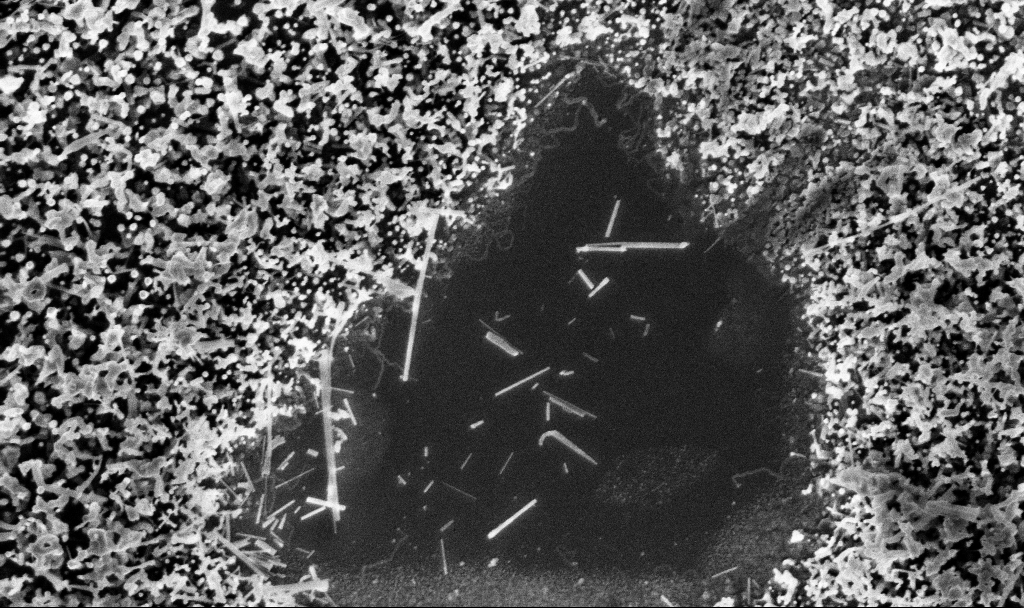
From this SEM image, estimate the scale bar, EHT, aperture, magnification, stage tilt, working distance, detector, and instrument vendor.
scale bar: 1000 nm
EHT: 3 kV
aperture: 30 µm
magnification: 51 K X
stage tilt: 0°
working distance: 3.1 mm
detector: InLens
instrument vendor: Zeiss